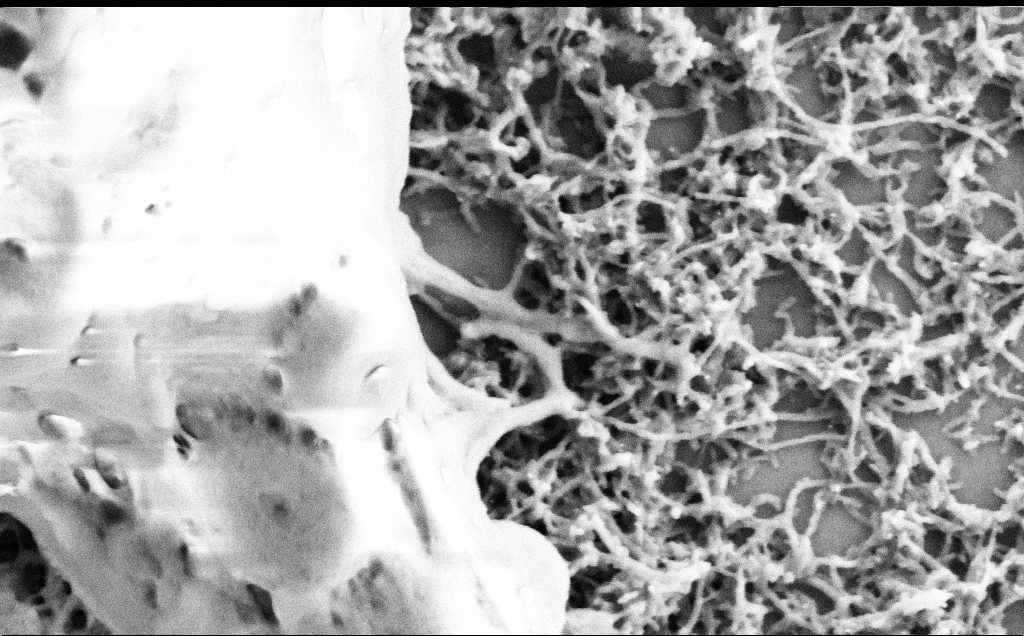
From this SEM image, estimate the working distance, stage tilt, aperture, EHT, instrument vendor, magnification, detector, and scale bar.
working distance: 7.1 mm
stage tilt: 0°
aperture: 30 µm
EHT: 2 kV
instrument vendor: Zeiss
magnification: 75 K X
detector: SE2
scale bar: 200 nm